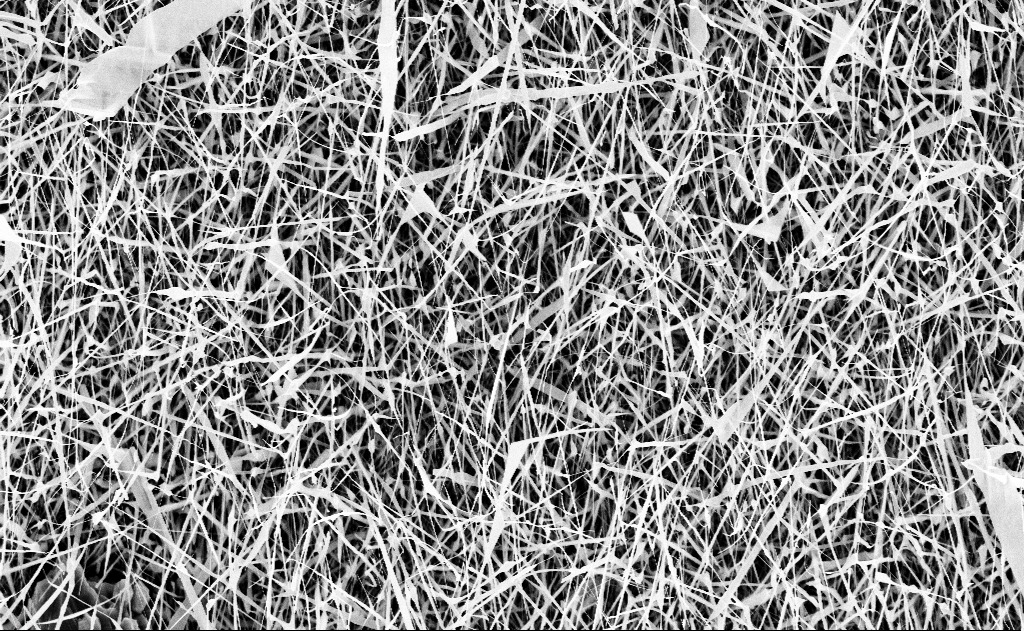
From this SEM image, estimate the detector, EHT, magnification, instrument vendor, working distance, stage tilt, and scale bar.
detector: InLens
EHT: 10 kV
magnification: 10 K X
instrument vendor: Zeiss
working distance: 16 mm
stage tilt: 0°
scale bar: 2000 nm